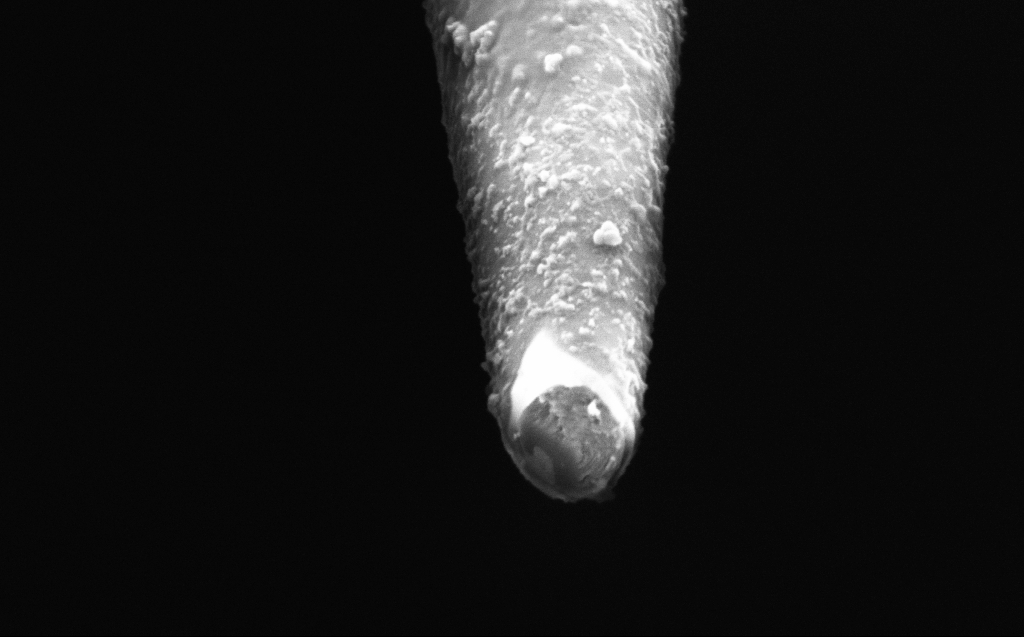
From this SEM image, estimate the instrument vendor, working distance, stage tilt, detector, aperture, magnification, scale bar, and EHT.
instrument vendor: Zeiss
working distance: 4 mm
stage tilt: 45°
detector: InLens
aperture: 30 µm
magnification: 100 K X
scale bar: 200 nm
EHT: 2 kV